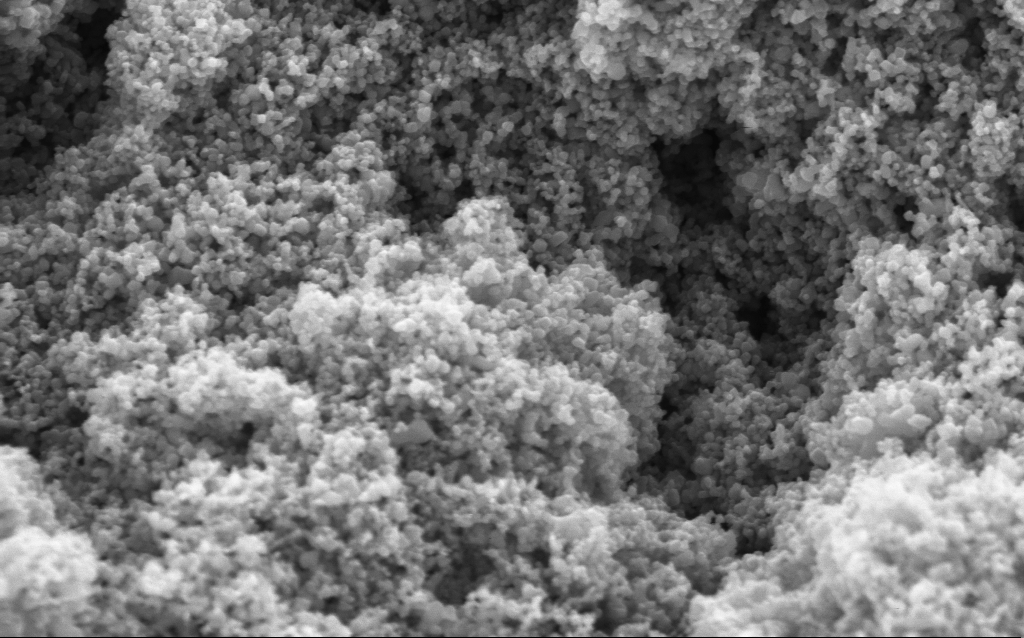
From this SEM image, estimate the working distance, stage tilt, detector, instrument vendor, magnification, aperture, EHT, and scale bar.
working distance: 4.5 mm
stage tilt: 0°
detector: InLens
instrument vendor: Zeiss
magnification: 114.65 K X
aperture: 30 µm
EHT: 5 kV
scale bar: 200 nm